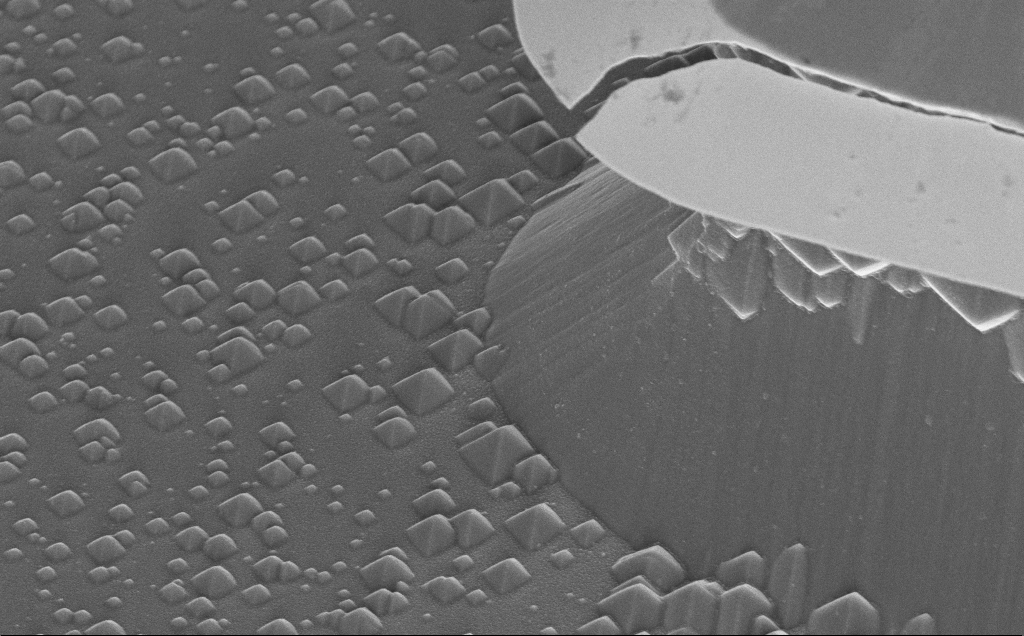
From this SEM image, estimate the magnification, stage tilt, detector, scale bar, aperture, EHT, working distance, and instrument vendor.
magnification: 12.59 K X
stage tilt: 45°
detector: InLens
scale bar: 2000 nm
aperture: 30 µm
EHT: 5 kV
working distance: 13 mm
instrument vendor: Zeiss